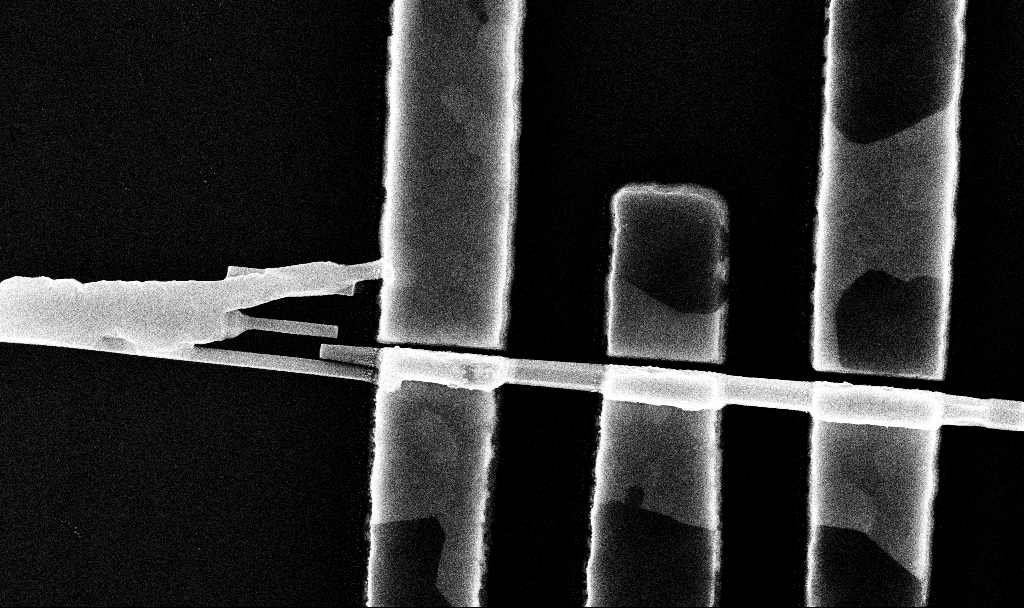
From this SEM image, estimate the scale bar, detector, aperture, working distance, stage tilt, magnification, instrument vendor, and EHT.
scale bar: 200 nm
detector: InLens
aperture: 30 µm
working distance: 6.8 mm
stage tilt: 0°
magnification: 75.74 K X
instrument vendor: Zeiss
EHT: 10 kV